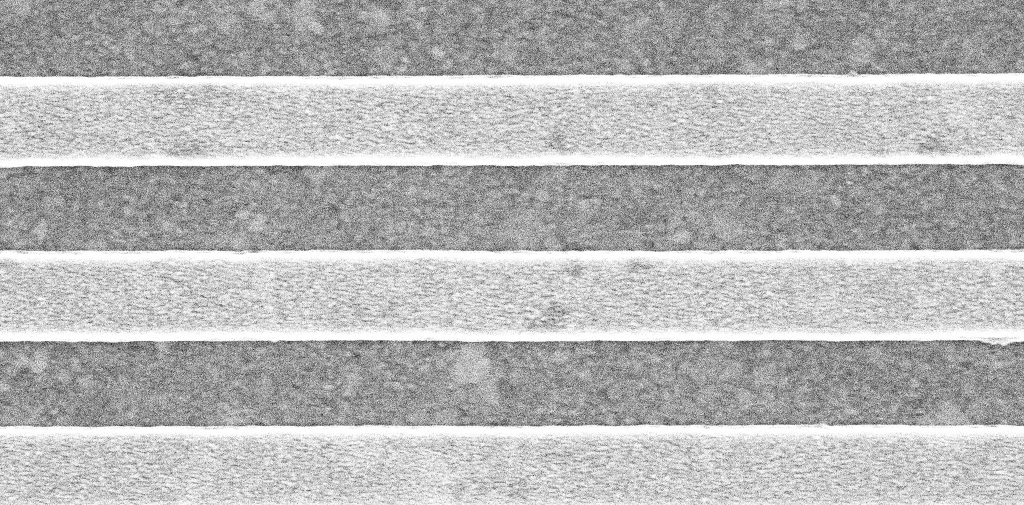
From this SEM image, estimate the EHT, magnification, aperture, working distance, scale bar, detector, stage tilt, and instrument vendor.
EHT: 5 kV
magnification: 80.96 K X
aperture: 30 µm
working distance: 3.1 mm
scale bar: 200 nm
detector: InLens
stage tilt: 0°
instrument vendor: Zeiss